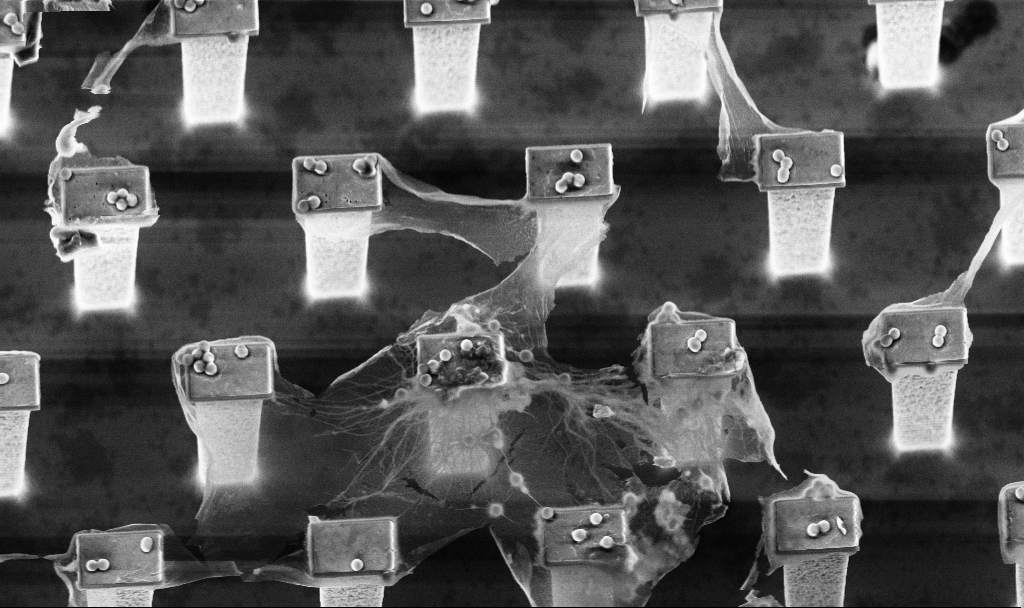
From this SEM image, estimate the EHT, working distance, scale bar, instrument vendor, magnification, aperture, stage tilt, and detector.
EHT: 5 kV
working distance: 3.2 mm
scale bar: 10000 nm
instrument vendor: Zeiss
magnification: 7.08 K X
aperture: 30 µm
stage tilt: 20°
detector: InLens